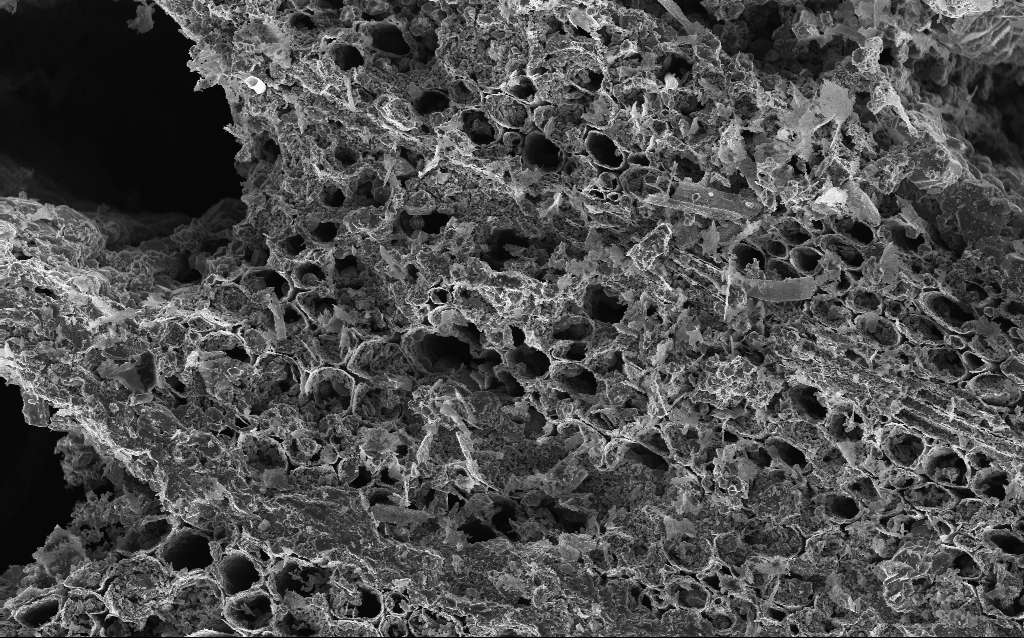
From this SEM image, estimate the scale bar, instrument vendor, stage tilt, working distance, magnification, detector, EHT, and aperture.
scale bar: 20000 nm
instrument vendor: Zeiss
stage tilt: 0°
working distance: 3 mm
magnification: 1 K X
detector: InLens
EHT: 10 kV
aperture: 30 µm